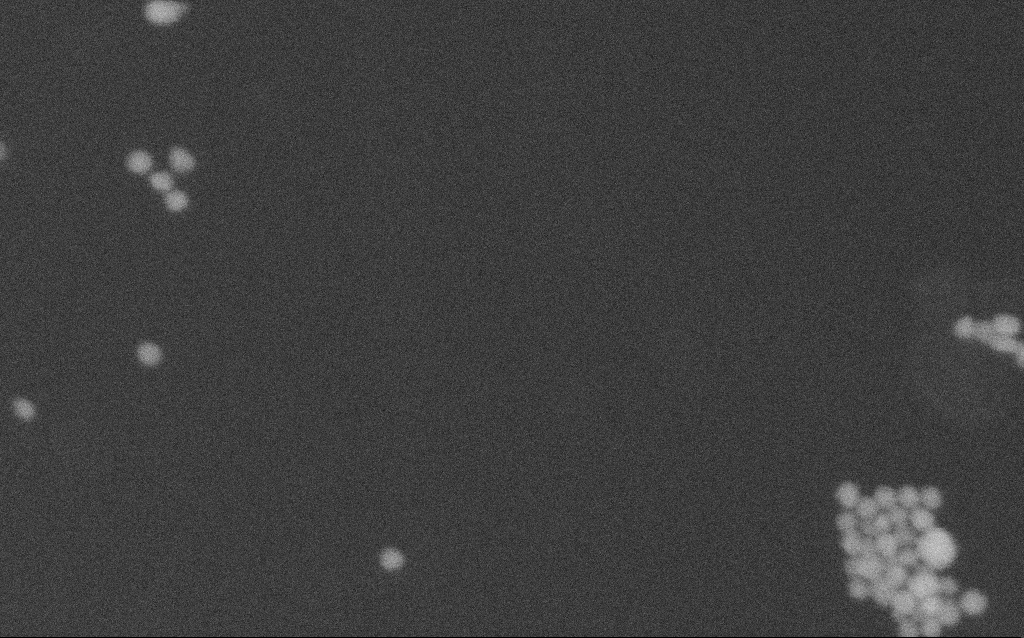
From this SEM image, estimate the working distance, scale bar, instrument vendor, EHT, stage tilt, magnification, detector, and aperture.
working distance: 2.1 mm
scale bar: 100 nm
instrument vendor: Zeiss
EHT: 10 kV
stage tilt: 0°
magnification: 577.71 K X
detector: SE2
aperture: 30 µm